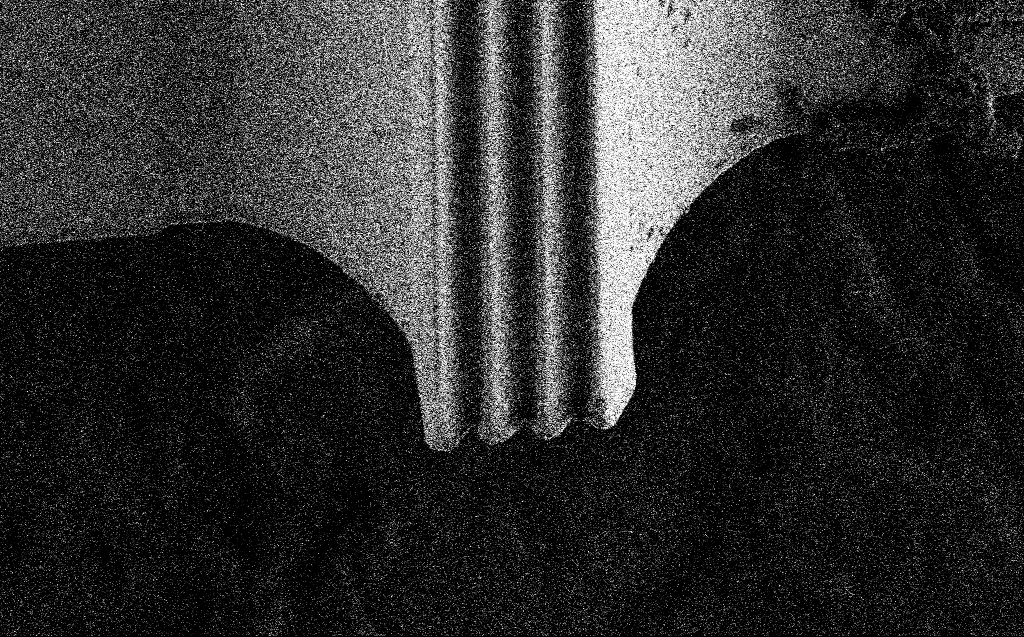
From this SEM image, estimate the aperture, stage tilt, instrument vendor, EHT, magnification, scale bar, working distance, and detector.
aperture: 30 µm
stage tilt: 44.6°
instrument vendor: Zeiss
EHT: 1 kV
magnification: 1.76 K X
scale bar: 10000 nm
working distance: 6 mm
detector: SE2